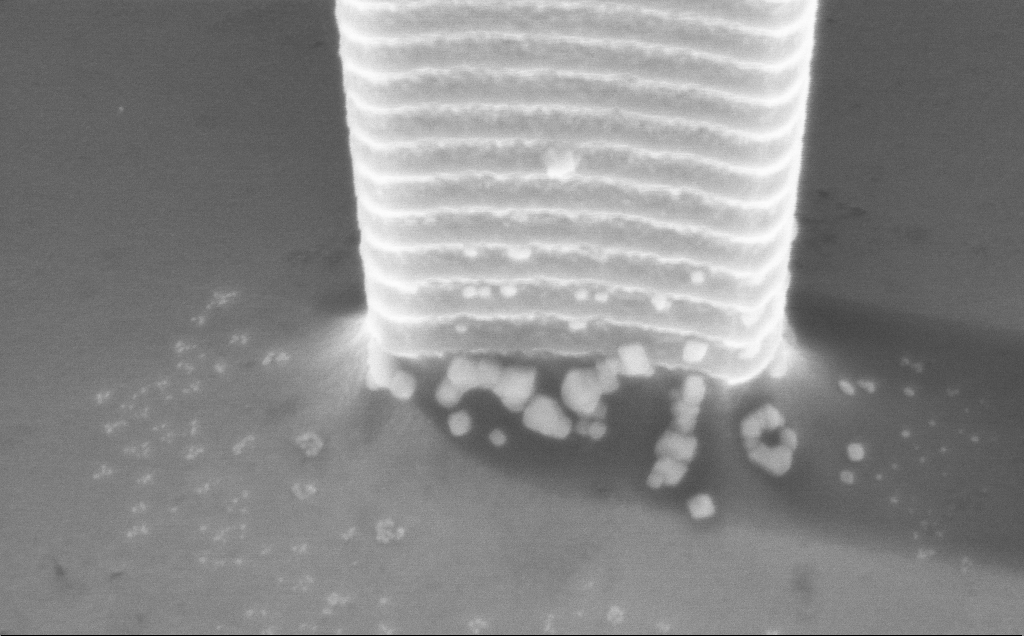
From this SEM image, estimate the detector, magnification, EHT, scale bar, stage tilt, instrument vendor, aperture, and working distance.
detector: InLens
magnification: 46.74 K X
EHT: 7.5 kV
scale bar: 1000 nm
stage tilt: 45°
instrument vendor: Zeiss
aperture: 30 µm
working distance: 5 mm